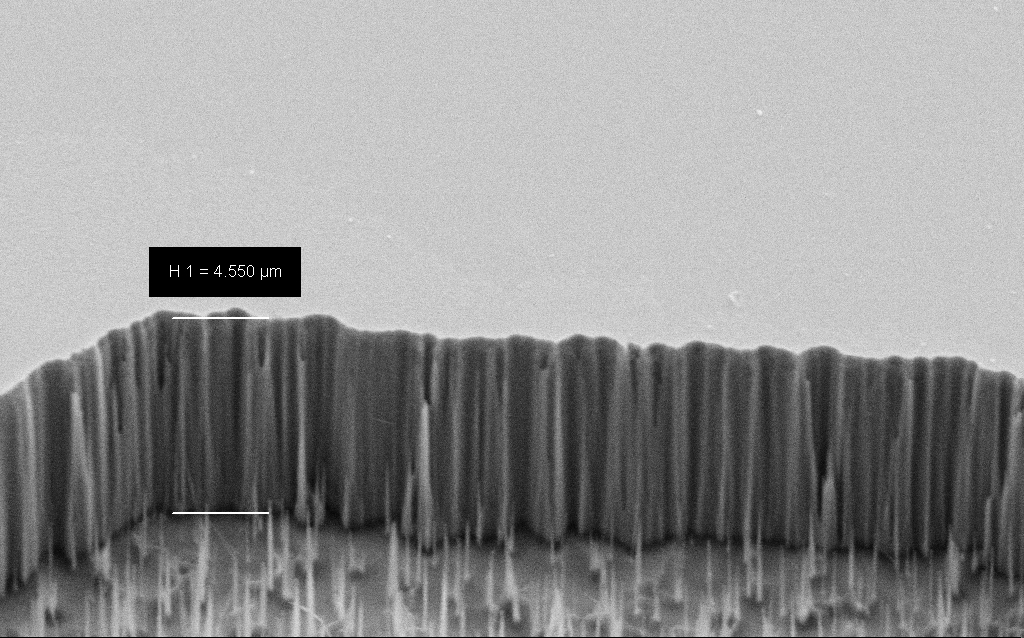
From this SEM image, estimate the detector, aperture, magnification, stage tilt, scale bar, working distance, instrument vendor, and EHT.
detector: SE2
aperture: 30 µm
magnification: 15.74 K X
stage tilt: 45°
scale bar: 1000 nm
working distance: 7 mm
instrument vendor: Zeiss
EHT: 5 kV